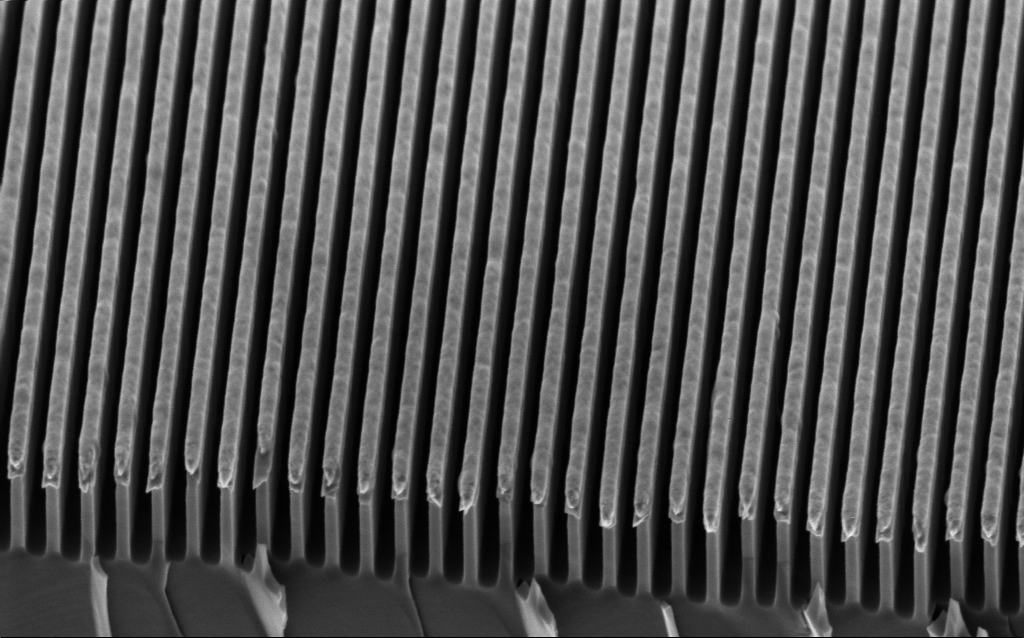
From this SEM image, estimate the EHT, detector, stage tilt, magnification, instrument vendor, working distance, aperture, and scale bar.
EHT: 2 kV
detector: InLens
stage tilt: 45°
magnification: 70.73 K X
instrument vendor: Zeiss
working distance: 3.2 mm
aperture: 30 µm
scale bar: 1000 nm